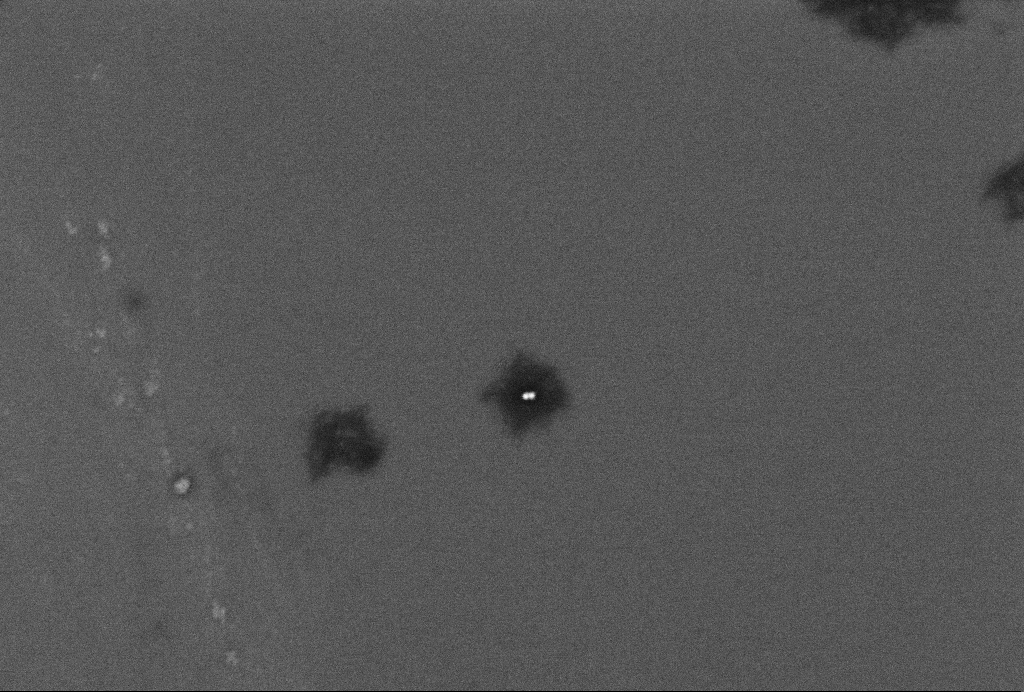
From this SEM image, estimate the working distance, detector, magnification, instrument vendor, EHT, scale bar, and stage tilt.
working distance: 3.3 mm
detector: InLens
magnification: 86.19 K X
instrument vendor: Zeiss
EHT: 2 kV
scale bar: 200 nm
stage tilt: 0°